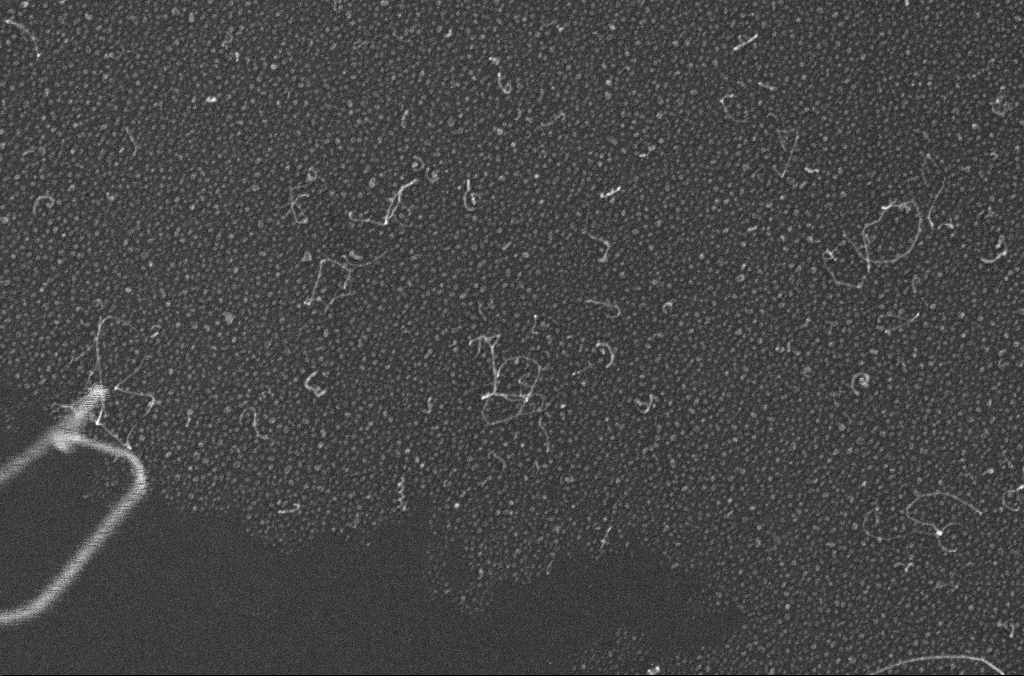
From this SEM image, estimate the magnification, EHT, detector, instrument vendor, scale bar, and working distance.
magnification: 100 K X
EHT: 10 kV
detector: InLens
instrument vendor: Zeiss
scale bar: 200 nm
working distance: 3 mm